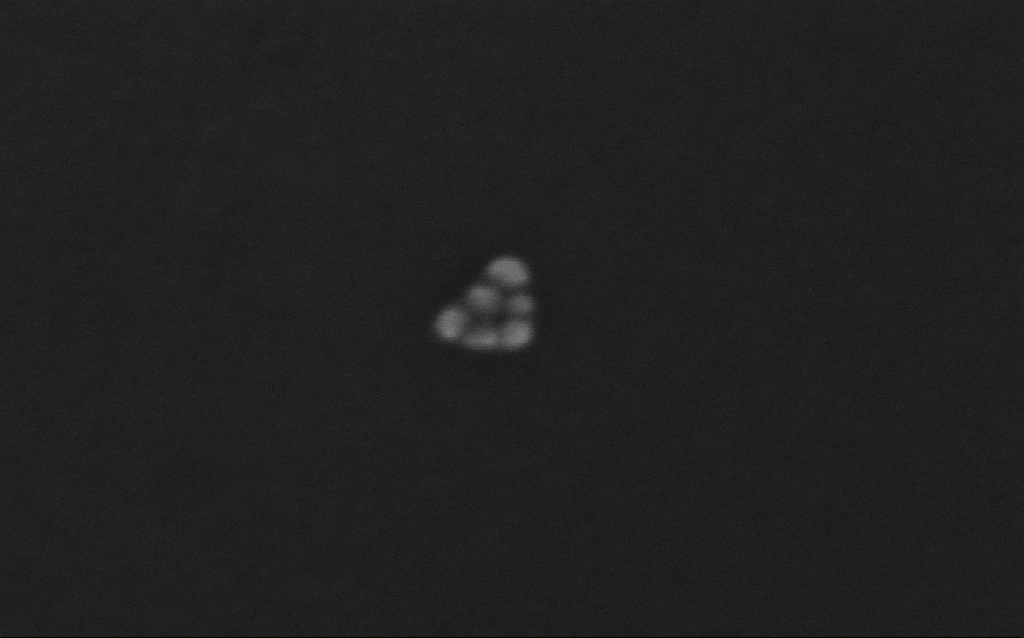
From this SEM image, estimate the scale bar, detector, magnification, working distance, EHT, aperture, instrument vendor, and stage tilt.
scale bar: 20 nm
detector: InLens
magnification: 776.18 K X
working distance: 7 mm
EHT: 10 kV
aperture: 30 µm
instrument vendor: Zeiss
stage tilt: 0°